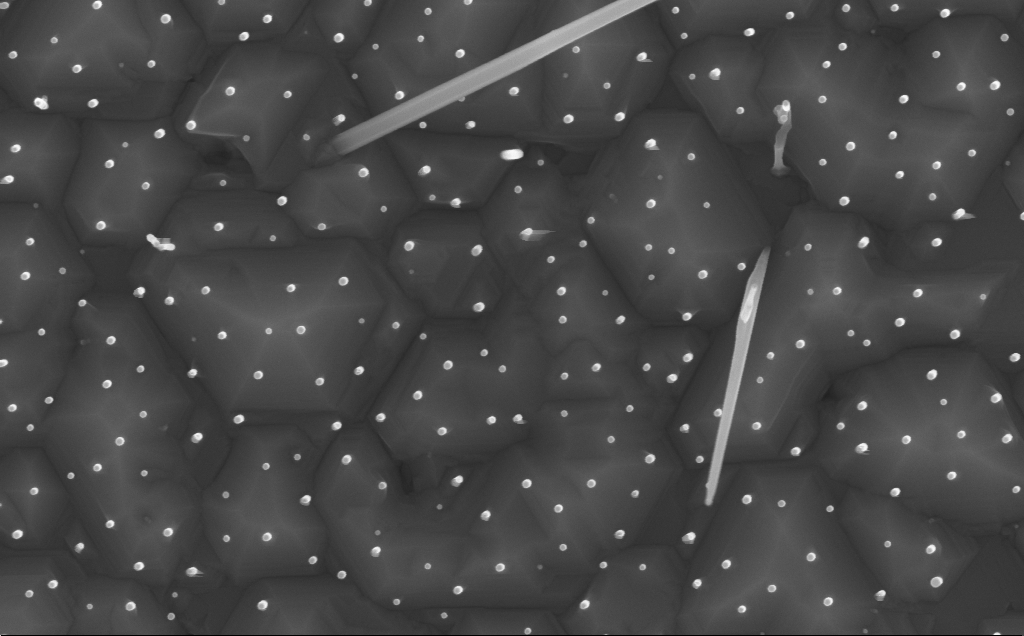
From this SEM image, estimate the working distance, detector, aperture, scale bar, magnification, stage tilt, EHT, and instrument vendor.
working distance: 7 mm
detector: InLens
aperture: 30 µm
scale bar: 200 nm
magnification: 80 K X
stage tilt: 0.2°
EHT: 10 kV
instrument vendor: Zeiss